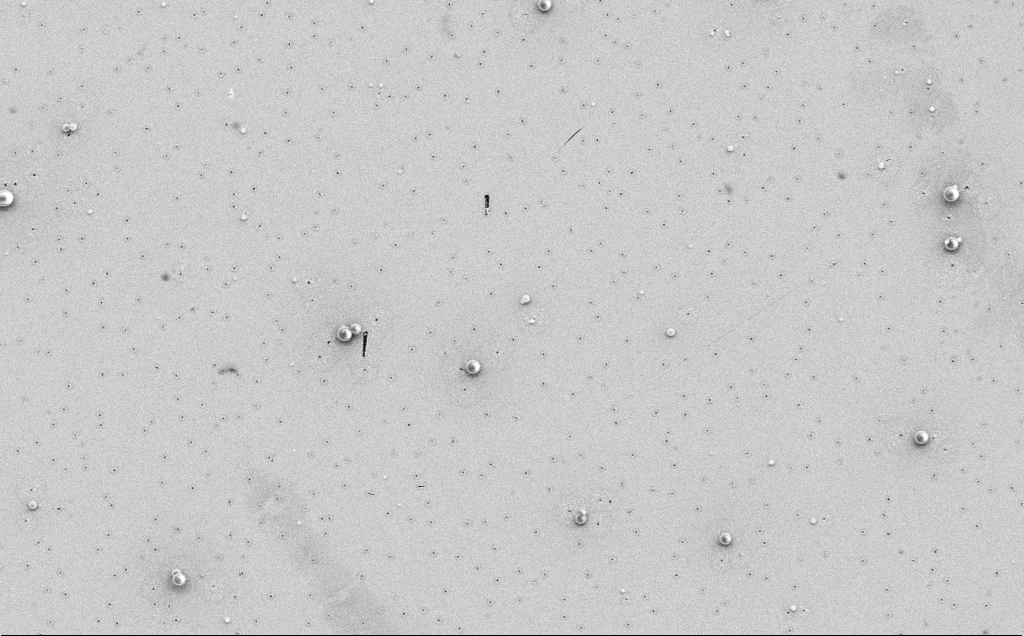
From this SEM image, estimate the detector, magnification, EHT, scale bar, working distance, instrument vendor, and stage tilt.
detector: SE2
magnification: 4.2 K X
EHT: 5 kV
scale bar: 10000 nm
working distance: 12 mm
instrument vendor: Zeiss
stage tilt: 0°